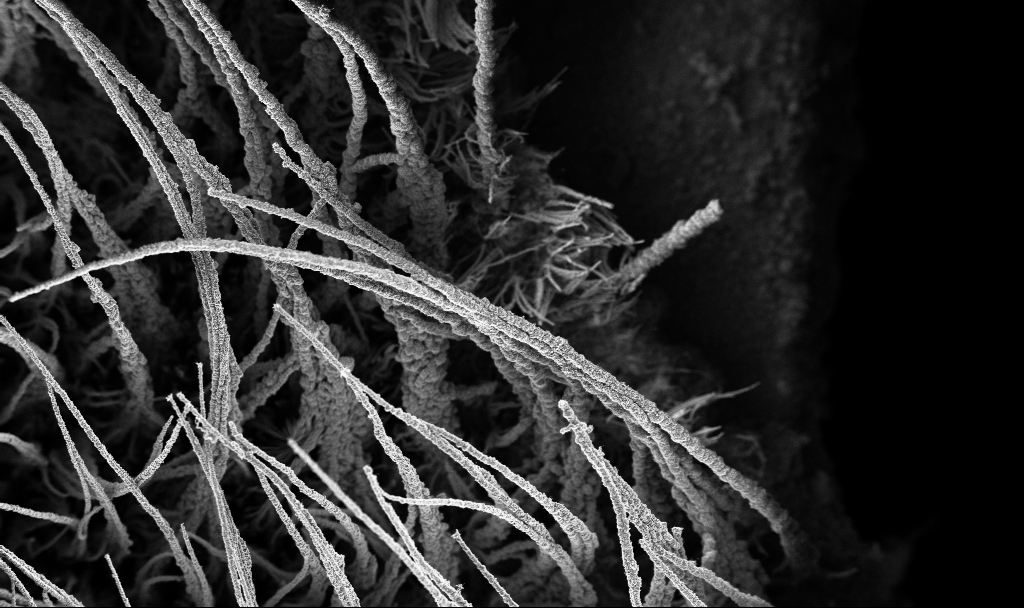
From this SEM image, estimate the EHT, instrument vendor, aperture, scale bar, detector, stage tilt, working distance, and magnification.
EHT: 3 kV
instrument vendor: Zeiss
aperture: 30 µm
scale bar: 100000 nm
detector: InLens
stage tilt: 0°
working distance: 2.9 mm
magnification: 0.15 K X